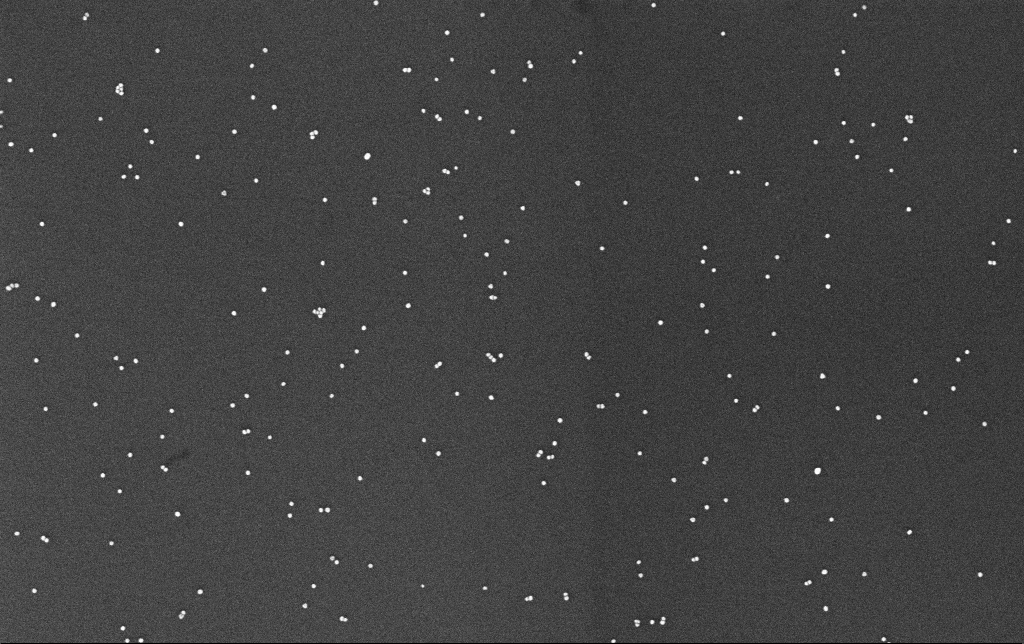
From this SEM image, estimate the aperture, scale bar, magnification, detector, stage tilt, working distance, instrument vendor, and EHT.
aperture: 30 µm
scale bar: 200 nm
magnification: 100 K X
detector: InLens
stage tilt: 0°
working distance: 3.3 mm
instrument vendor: Zeiss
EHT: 10 kV